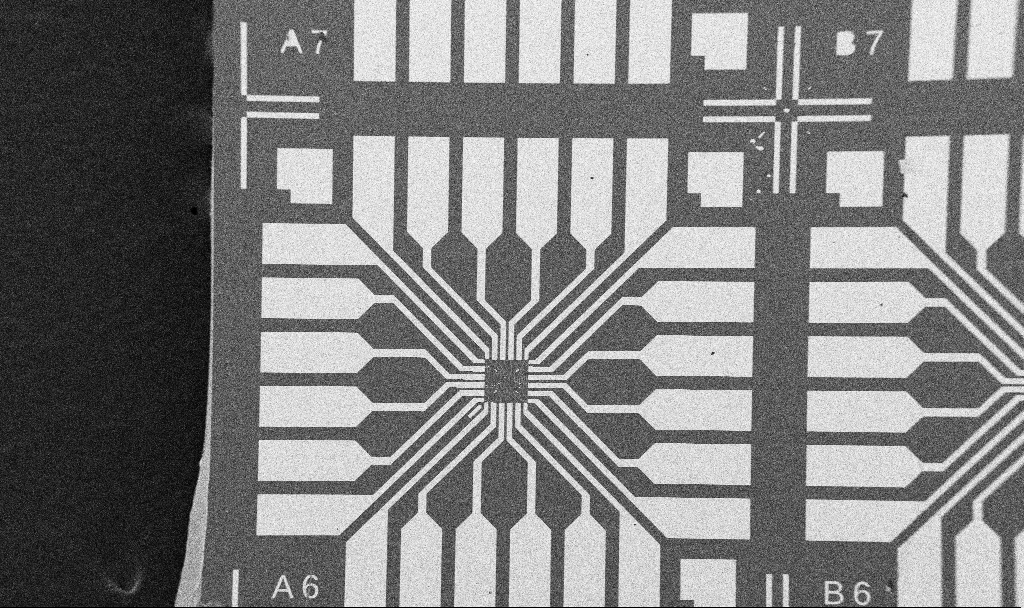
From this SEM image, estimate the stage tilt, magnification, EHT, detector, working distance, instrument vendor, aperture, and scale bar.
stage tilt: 0°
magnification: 0.1 K X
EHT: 5 kV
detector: SE2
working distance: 10.7 mm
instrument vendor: Zeiss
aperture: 30 µm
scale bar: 200000 nm